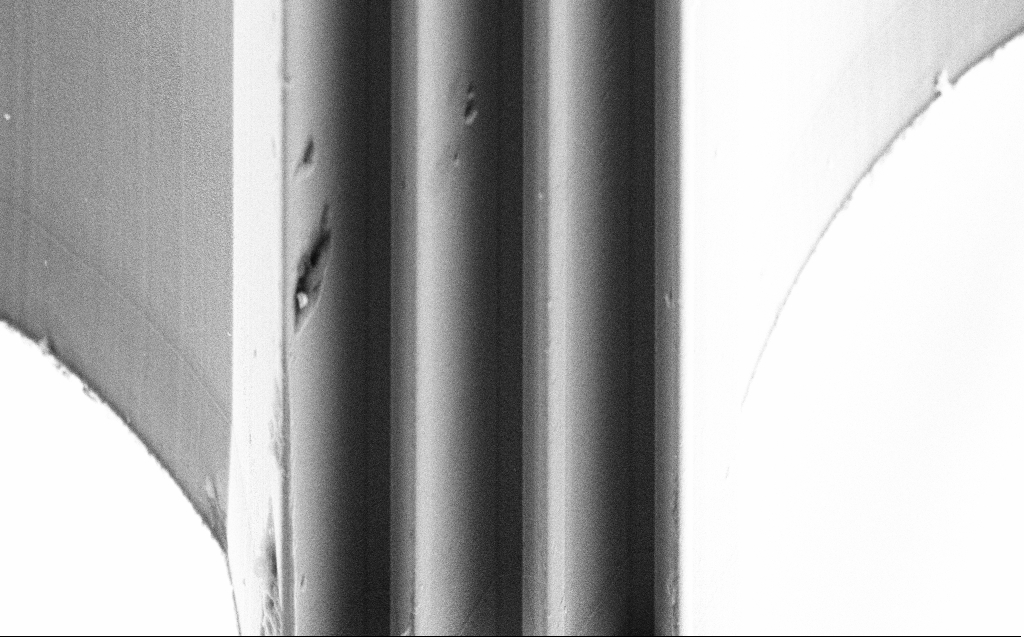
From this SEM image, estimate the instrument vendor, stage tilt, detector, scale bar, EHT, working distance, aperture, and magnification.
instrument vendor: Zeiss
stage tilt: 45°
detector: SE2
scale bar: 10000 nm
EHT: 10 kV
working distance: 9 mm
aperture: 30 µm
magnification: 4.33 K X